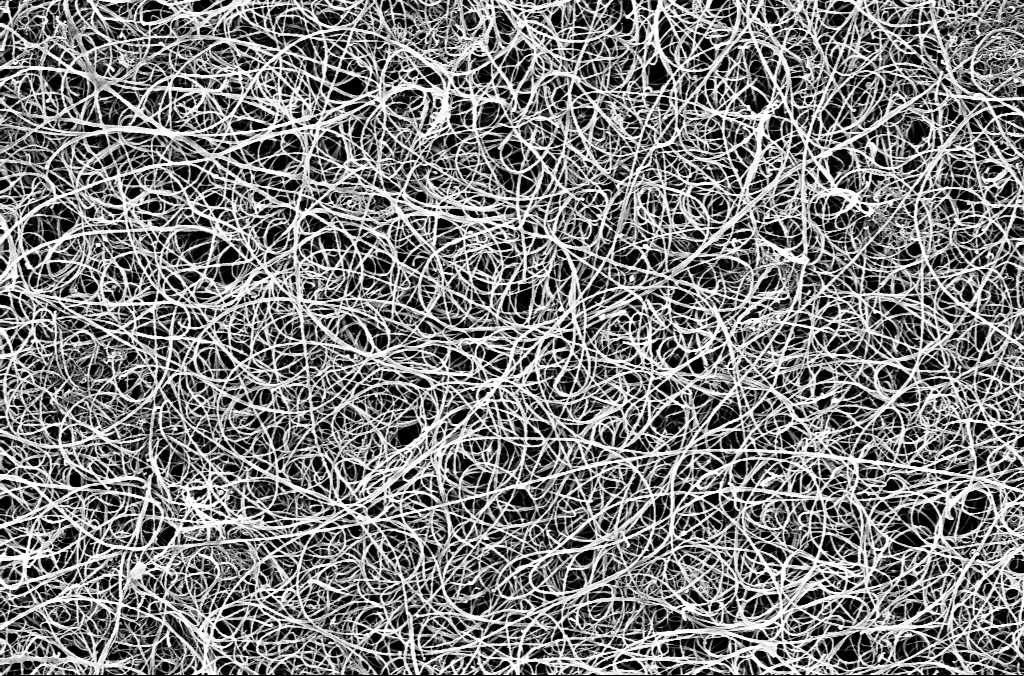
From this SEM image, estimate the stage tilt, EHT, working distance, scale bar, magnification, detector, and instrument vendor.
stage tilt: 0°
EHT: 5 kV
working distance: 3 mm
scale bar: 2000 nm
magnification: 25 K X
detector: InLens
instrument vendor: Zeiss